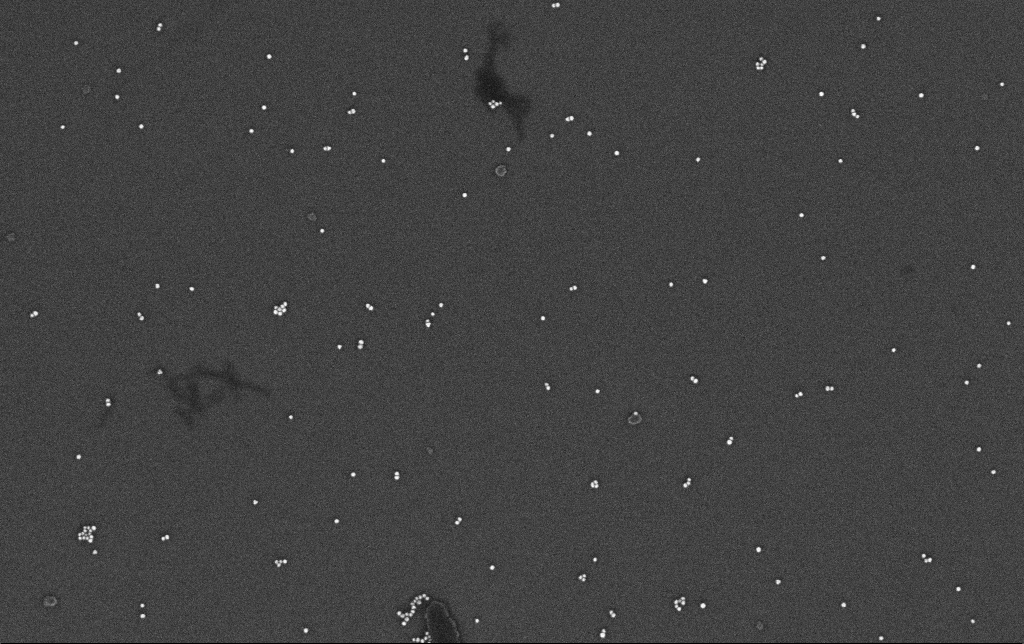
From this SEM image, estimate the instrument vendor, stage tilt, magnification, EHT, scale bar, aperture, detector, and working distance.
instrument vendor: Zeiss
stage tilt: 0°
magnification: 100 K X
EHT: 10 kV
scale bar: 200 nm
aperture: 30 µm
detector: InLens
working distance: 3.3 mm